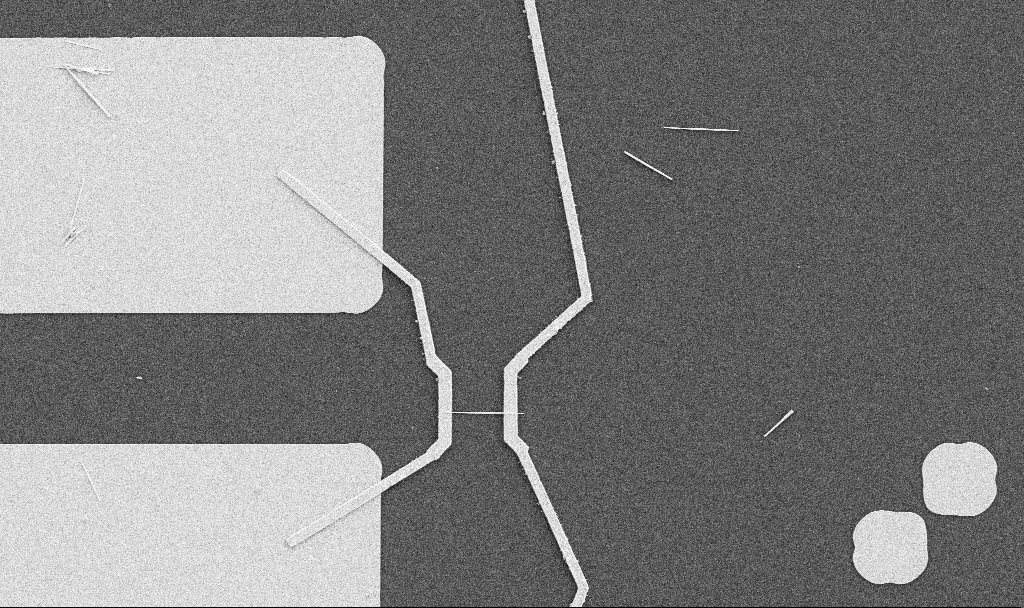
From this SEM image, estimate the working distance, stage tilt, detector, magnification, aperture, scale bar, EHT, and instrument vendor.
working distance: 10.7 mm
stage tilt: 0°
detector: SE2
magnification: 5 K X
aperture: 30 µm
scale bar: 10000 nm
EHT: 5 kV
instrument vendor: Zeiss